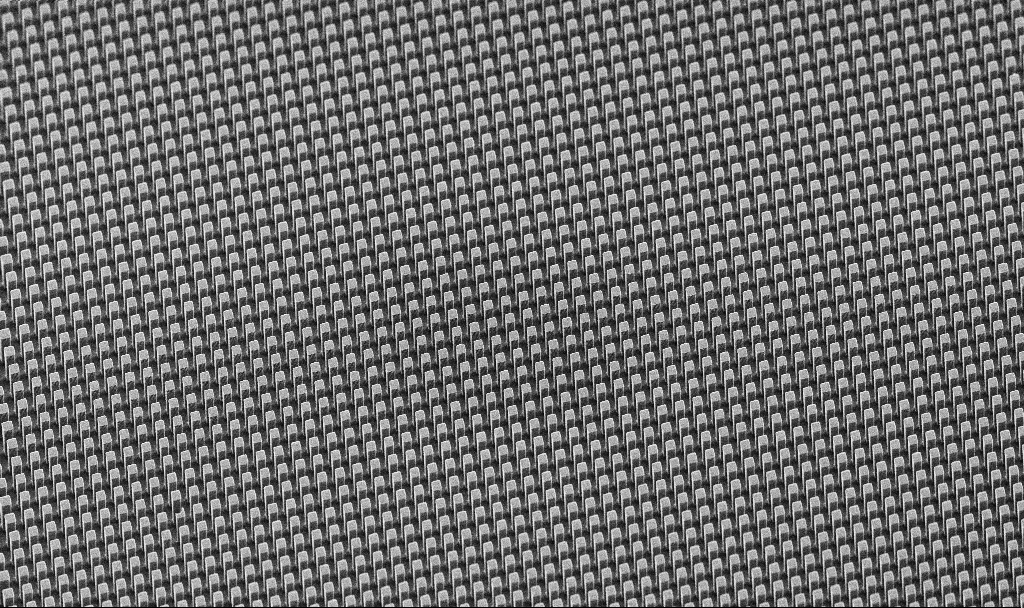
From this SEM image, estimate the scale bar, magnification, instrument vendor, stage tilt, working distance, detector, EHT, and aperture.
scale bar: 20000 nm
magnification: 0.832 K X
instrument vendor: Zeiss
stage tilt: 45°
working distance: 10 mm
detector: SE2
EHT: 5 kV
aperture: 30 µm